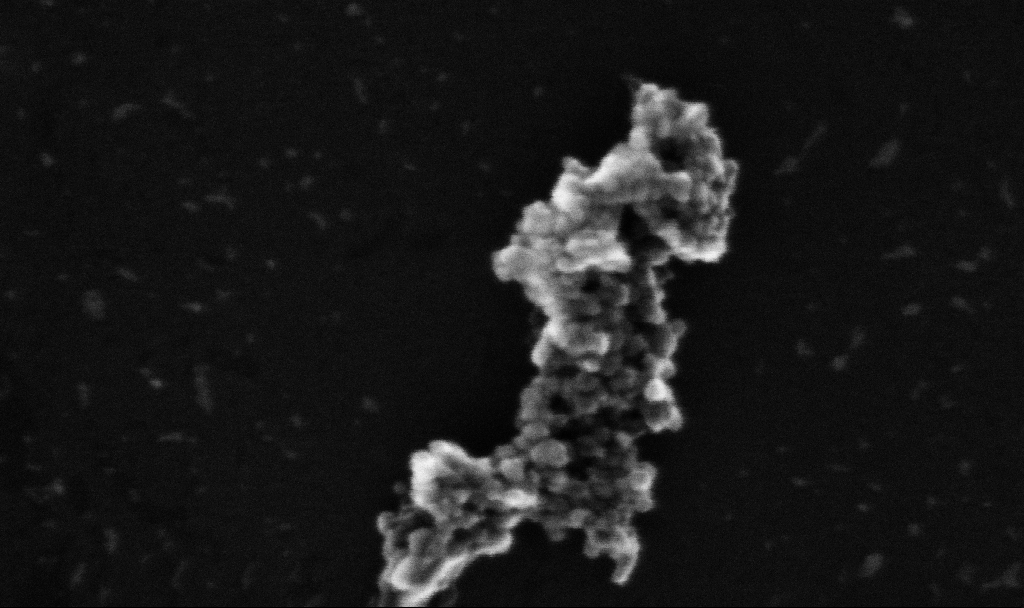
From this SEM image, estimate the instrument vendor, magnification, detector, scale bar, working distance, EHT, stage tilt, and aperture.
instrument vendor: Zeiss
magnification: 457.8 K X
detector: InLens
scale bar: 100 nm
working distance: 5.4 mm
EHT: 10 kV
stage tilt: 0°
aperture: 30 µm